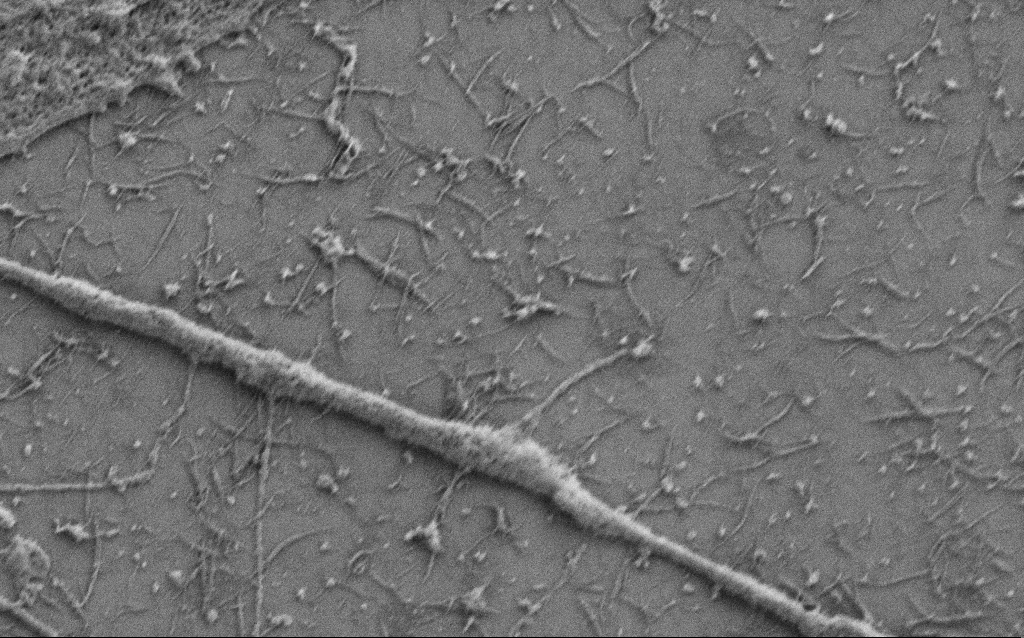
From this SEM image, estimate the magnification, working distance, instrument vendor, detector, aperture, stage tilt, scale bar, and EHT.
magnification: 25 K X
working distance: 5.7 mm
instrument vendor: Zeiss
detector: SE2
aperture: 30 µm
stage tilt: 0°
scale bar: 2000 nm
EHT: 0.8 kV